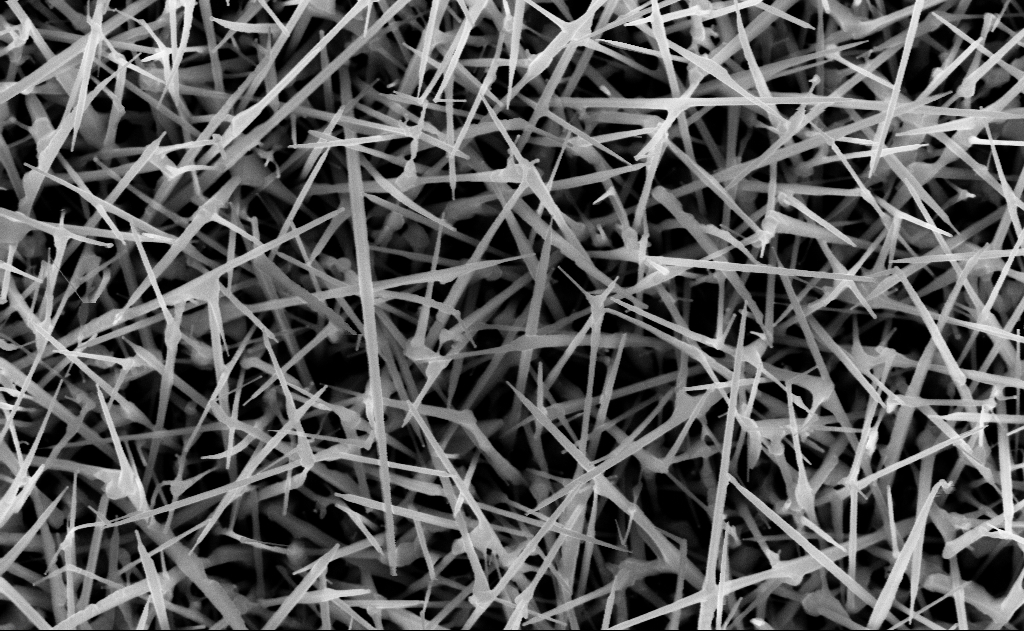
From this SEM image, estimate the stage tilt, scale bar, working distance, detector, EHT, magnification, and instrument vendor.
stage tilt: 0°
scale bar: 1000 nm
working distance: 13 mm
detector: InLens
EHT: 10 kV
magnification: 40 K X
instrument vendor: Zeiss